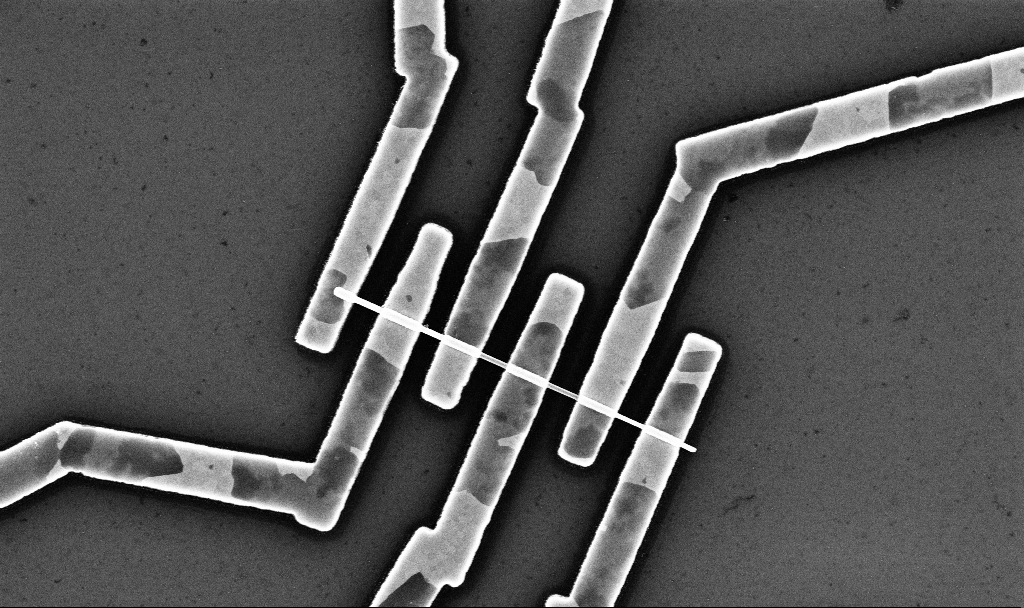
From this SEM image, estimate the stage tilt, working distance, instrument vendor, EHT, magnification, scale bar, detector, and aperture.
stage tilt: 0°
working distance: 6.8 mm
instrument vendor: Zeiss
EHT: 10 kV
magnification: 23.26 K X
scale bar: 2000 nm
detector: InLens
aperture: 30 µm